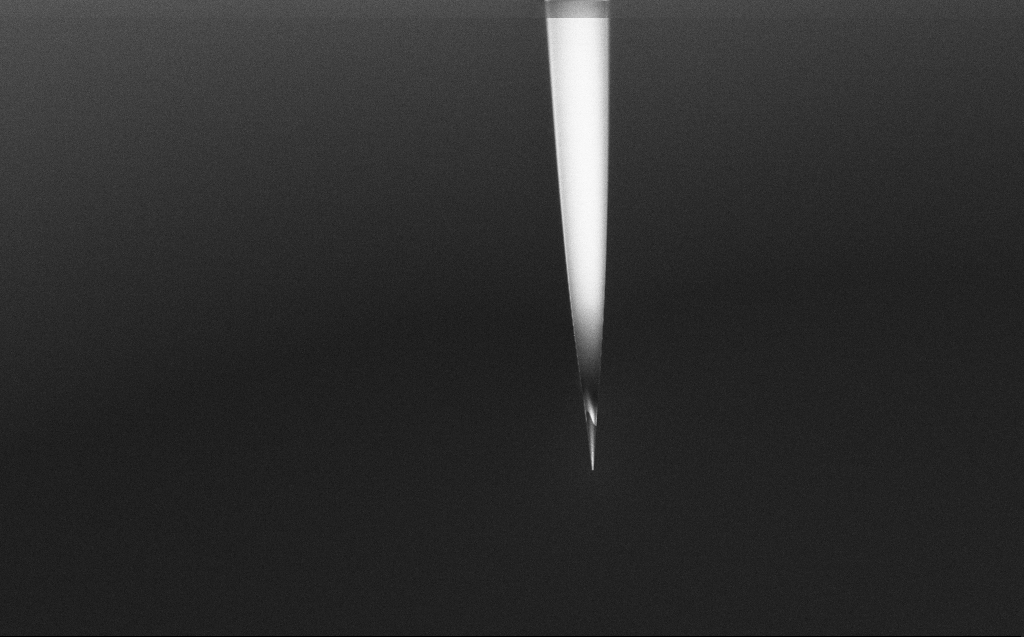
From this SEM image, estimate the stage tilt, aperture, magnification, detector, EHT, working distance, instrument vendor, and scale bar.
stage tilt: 45°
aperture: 30 µm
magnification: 1 K X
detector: InLens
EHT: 2 kV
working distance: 6 mm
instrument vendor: Zeiss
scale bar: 20000 nm